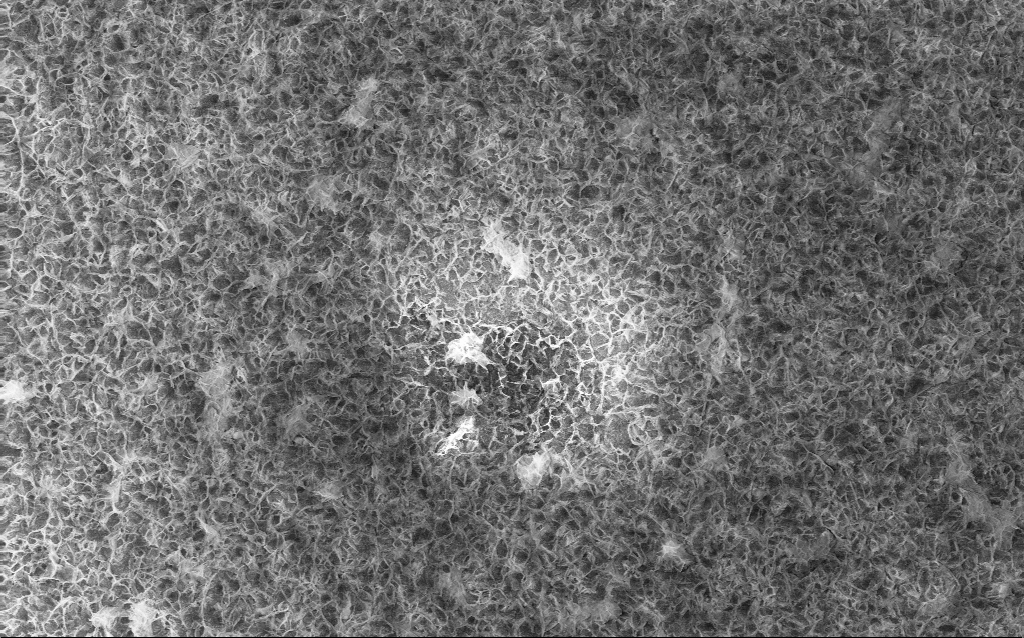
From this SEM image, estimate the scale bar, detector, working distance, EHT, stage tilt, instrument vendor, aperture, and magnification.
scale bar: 10000 nm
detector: InLens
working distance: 2.8 mm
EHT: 10 kV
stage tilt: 0°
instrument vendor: Zeiss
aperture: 30 µm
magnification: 4 K X